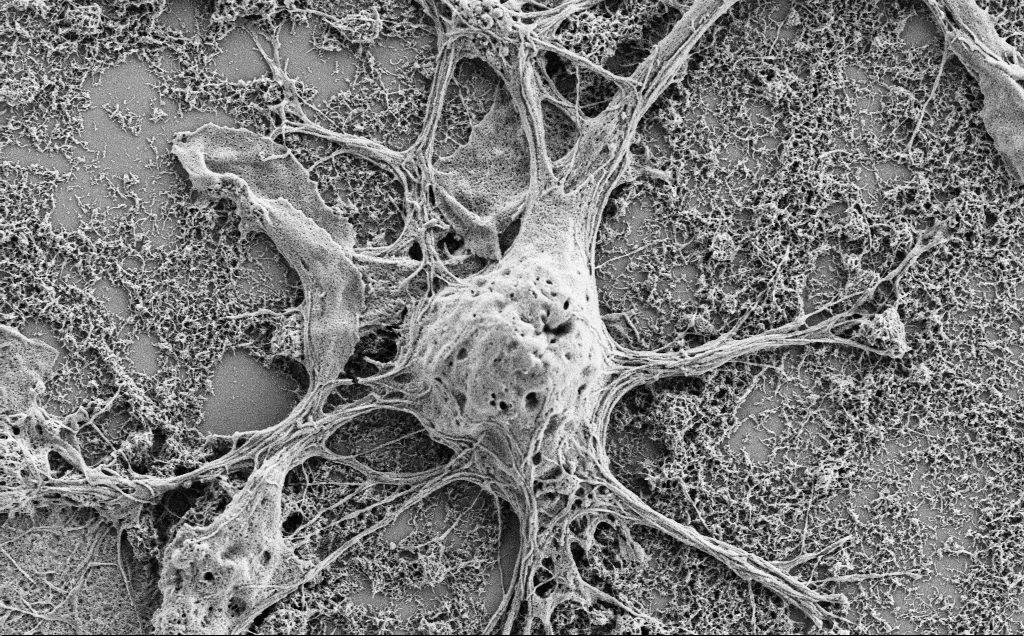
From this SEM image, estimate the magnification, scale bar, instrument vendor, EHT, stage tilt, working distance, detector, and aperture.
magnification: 10 K X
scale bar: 2000 nm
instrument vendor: Zeiss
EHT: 2 kV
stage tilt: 0°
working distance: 7 mm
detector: SE2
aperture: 30 µm